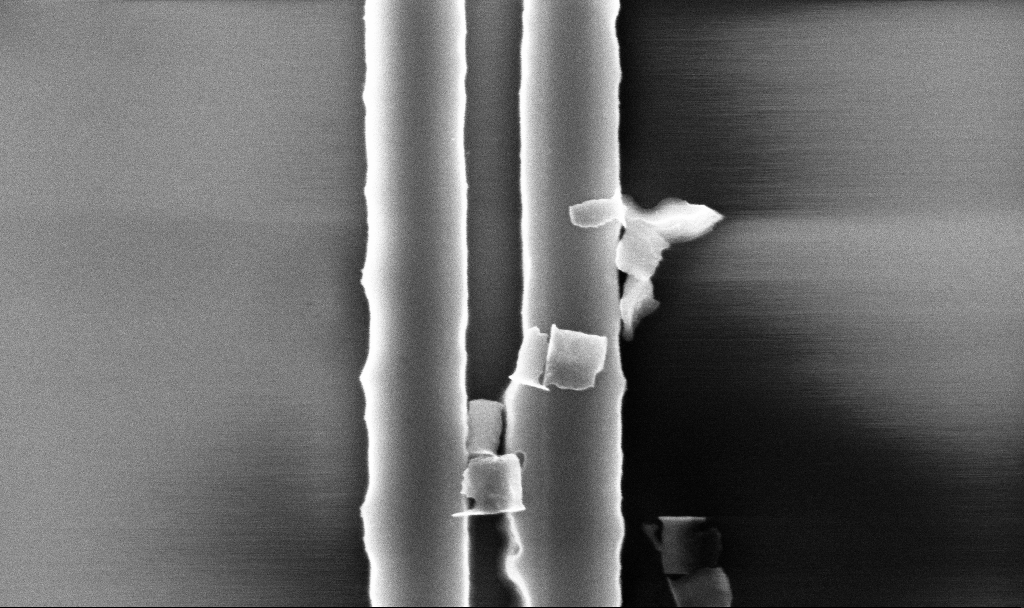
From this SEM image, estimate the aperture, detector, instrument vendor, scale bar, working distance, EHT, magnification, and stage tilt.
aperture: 30 µm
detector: InLens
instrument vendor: Zeiss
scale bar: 200 nm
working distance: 5.2 mm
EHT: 5 kV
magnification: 78.47 K X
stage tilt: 0°